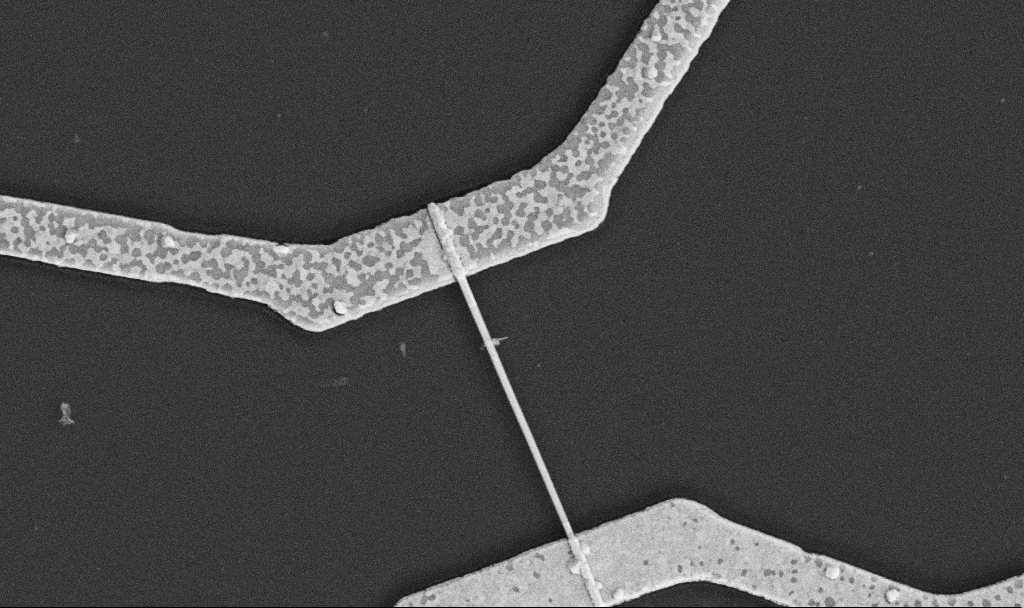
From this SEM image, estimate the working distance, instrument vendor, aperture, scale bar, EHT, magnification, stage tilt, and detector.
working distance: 10.6 mm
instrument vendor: Zeiss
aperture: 30 µm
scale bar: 1000 nm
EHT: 5 kV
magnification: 30 K X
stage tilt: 0°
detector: SE2